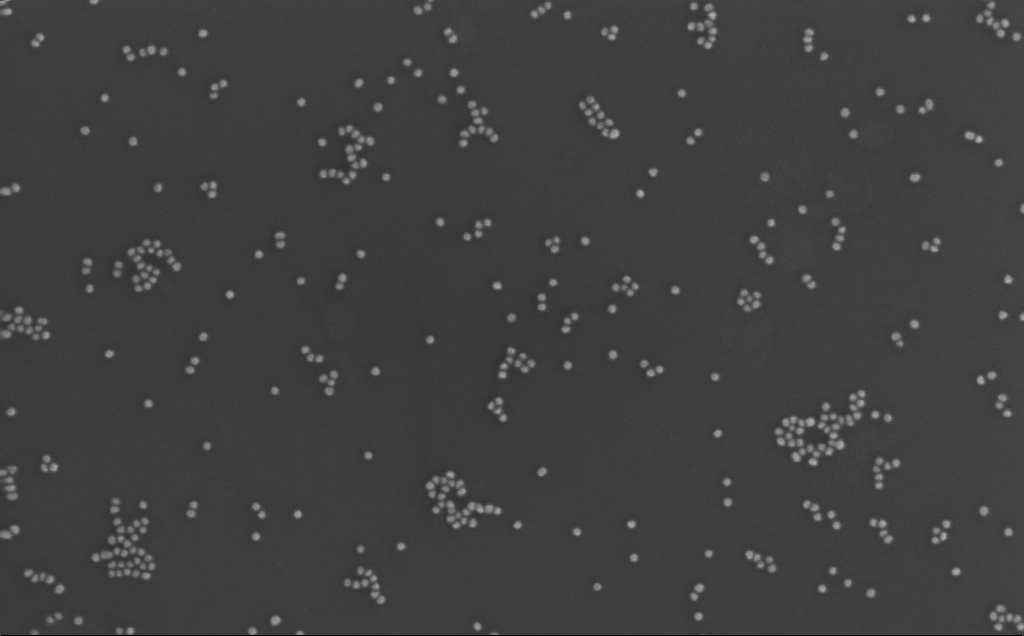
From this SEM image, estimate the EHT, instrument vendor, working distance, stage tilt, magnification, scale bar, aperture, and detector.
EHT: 10 kV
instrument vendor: Zeiss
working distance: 3 mm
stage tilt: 0°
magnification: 172.63 K X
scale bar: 200 nm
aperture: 30 µm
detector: InLens